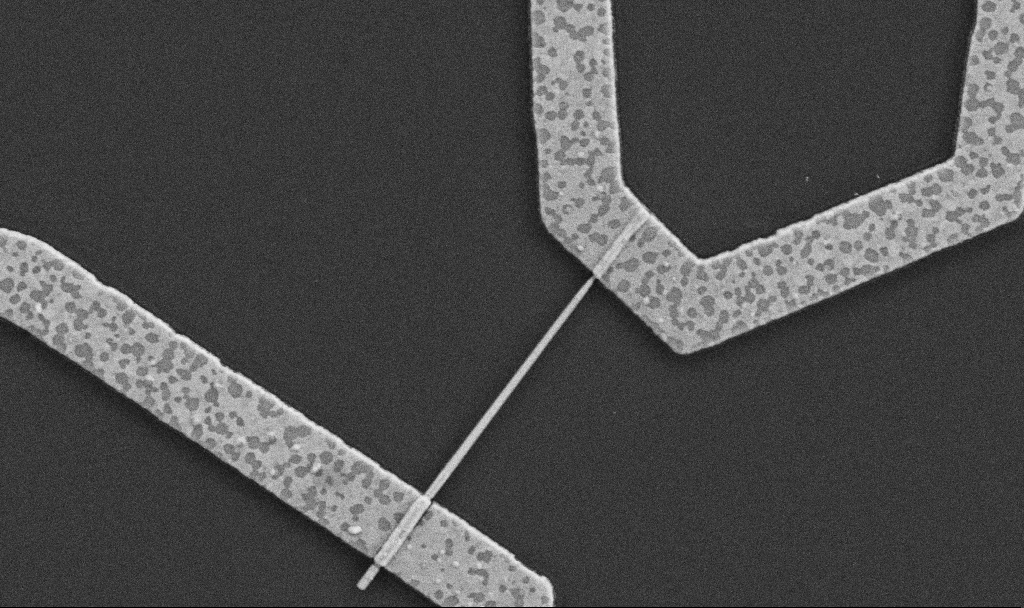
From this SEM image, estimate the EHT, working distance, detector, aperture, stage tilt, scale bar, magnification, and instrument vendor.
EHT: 5 kV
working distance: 8.7 mm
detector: SE2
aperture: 30 µm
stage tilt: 0°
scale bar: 1000 nm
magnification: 30 K X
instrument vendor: Zeiss